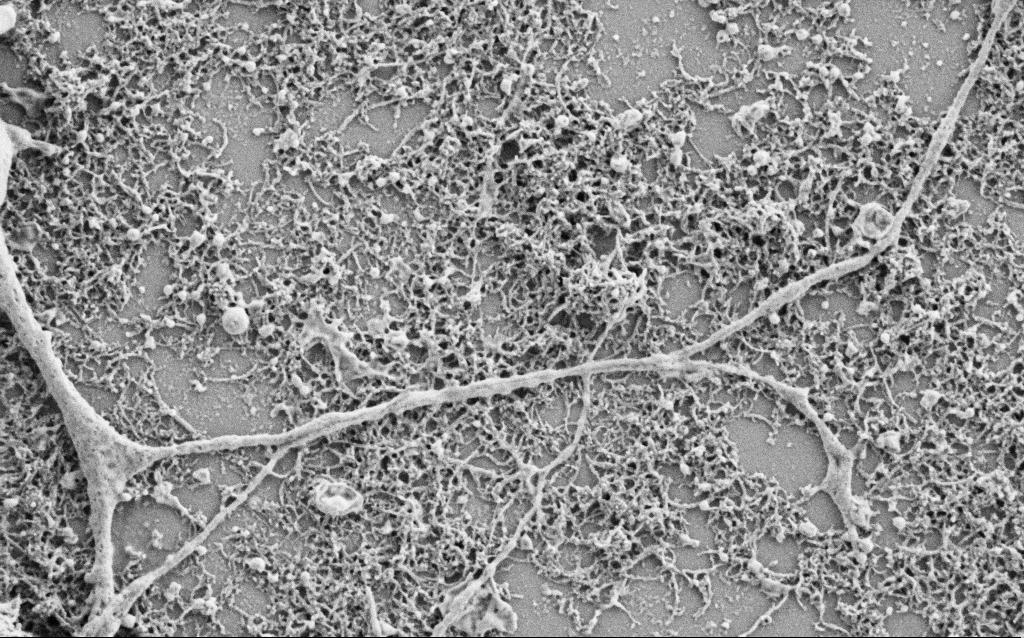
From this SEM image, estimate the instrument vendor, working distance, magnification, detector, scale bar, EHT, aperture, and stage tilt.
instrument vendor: Zeiss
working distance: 6.8 mm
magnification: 25 K X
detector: SE2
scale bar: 1000 nm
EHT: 1.5 kV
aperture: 30 µm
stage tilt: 0°